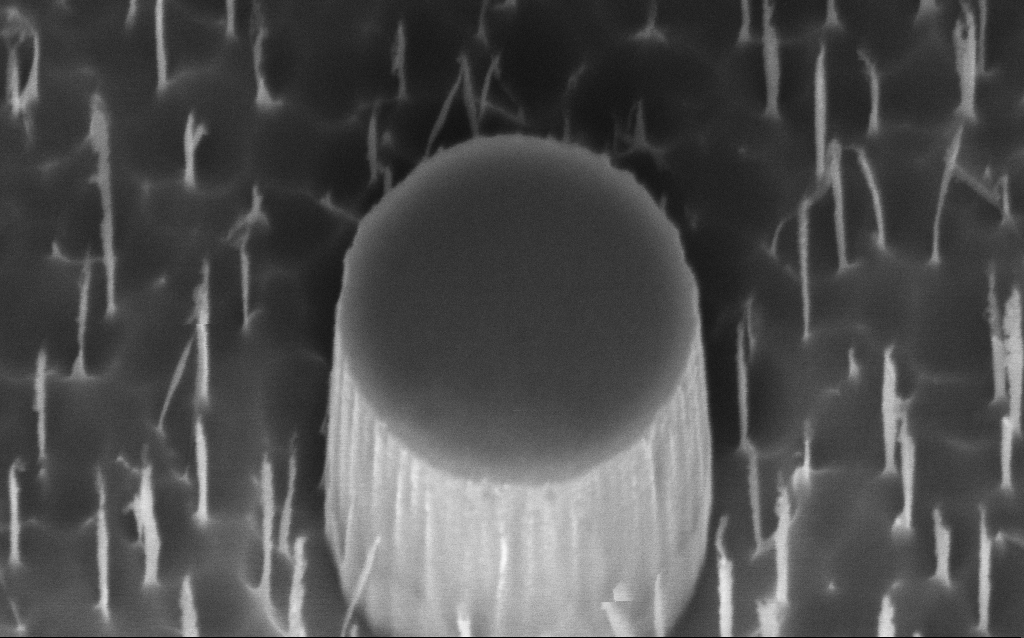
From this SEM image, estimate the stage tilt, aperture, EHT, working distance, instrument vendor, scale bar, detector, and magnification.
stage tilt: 45°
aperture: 30 µm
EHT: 3 kV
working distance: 8 mm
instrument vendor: Zeiss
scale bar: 200 nm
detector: InLens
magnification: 136.33 K X